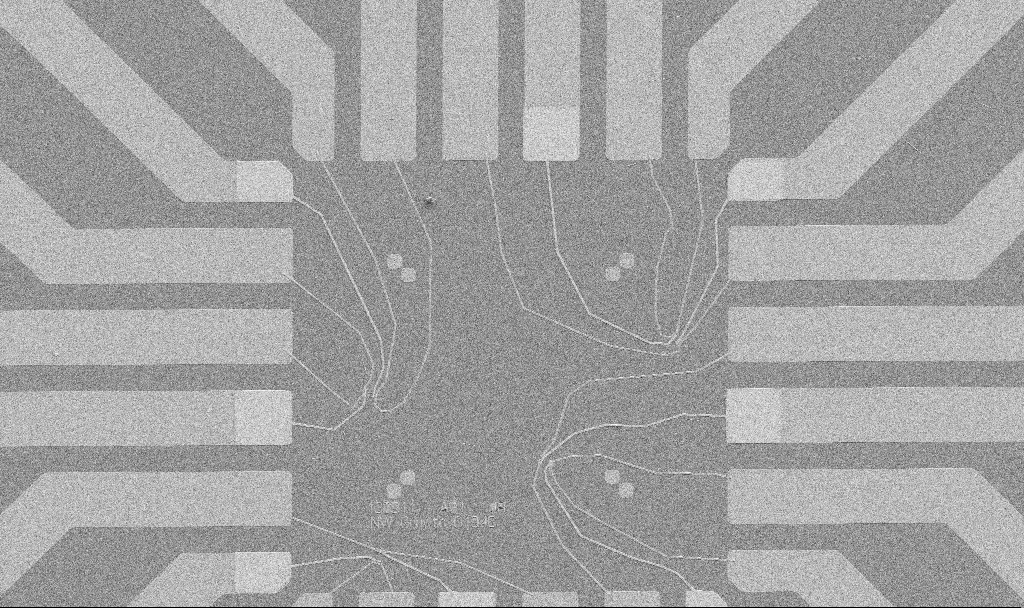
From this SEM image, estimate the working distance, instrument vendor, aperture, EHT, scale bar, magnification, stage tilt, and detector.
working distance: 10.7 mm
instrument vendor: Zeiss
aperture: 30 µm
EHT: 5 kV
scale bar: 20000 nm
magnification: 1 K X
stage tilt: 0°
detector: SE2